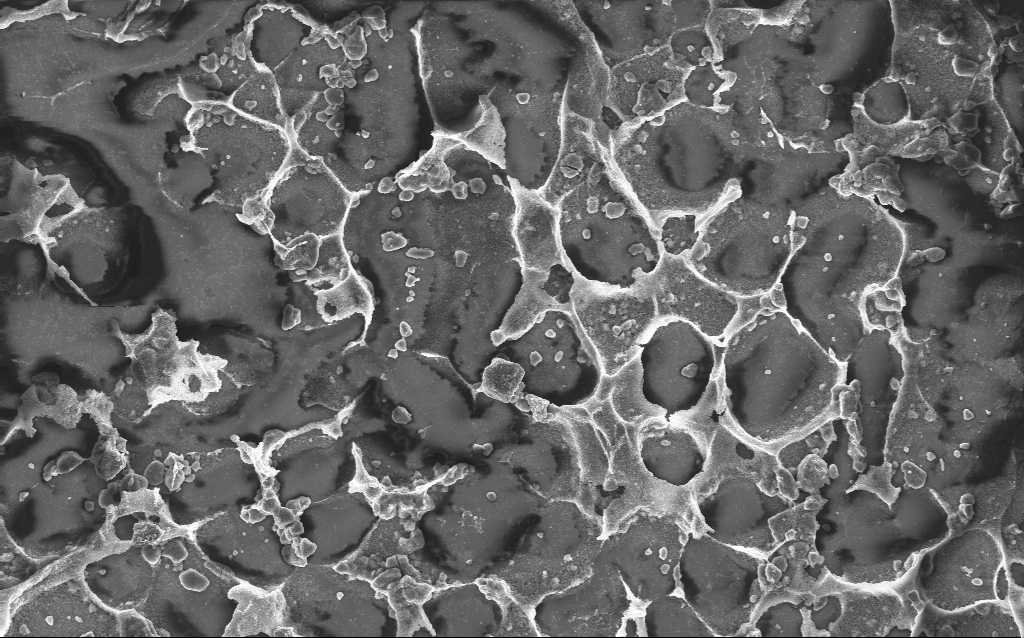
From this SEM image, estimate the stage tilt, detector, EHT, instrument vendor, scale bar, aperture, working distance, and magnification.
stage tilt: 0°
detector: InLens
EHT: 10 kV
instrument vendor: Zeiss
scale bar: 10000 nm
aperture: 30 µm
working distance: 2.8 mm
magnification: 1.69 K X